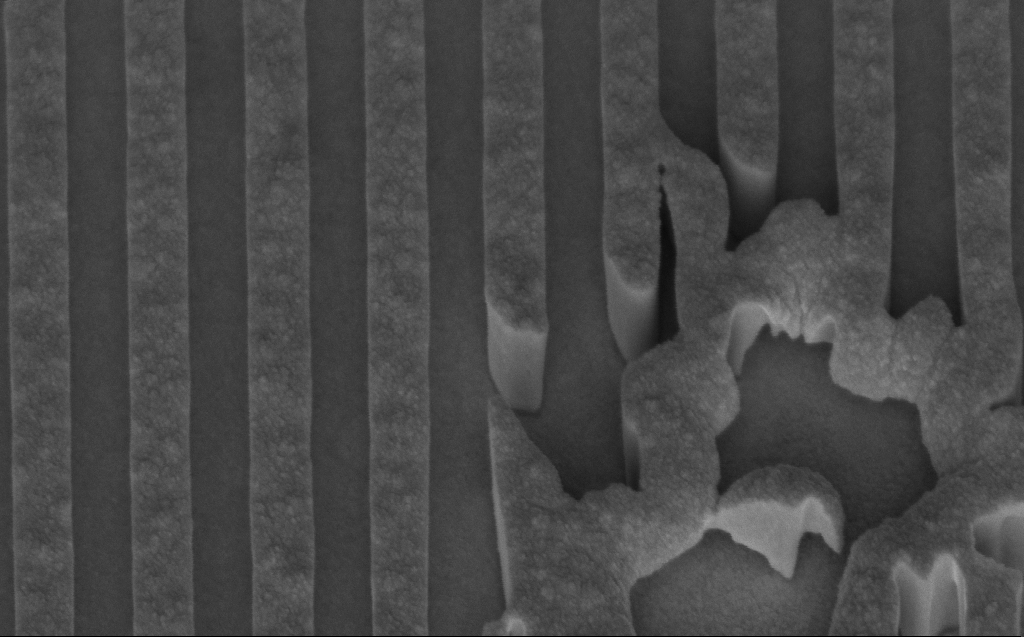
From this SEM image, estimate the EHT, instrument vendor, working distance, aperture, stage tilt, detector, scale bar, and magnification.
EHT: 5 kV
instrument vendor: Zeiss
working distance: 8 mm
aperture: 30 µm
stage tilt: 45°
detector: InLens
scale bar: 200 nm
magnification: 87.55 K X